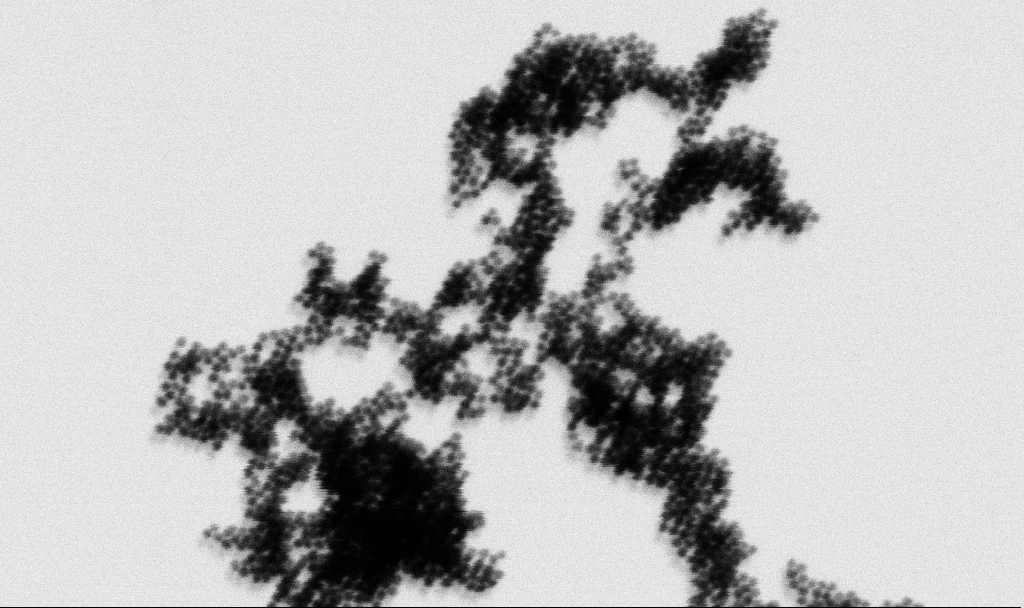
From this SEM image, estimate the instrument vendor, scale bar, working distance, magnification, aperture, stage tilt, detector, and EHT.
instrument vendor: Zeiss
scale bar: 100 nm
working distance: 5 mm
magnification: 200 K X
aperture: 30 µm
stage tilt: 0°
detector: SE2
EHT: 3 kV